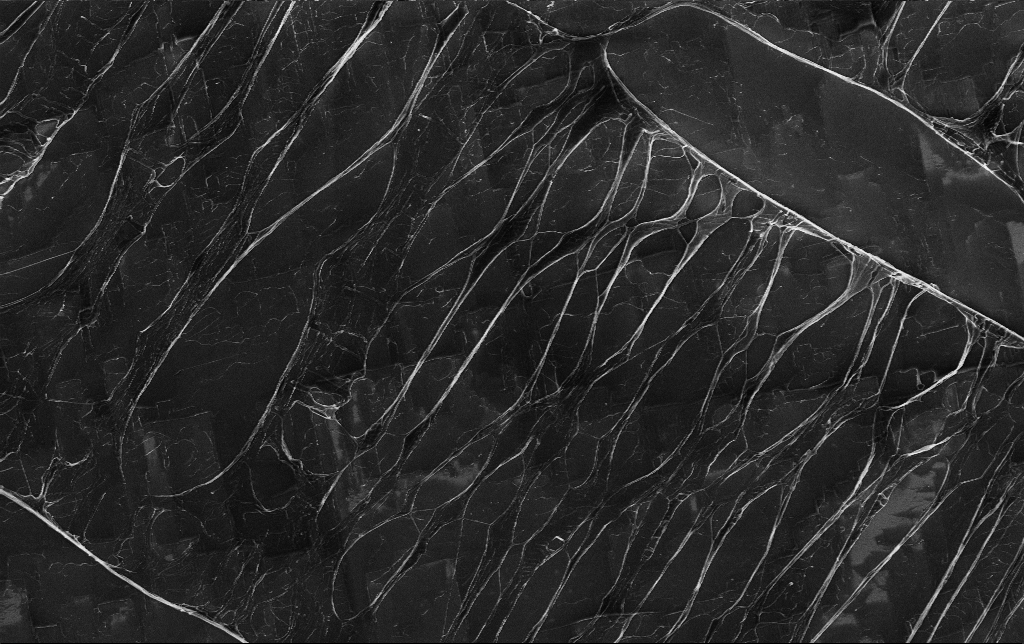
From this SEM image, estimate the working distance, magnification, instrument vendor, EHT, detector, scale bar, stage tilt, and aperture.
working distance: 3.8 mm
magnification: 2.78 K X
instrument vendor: Zeiss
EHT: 5 kV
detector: InLens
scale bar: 10000 nm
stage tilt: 0°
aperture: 30 µm